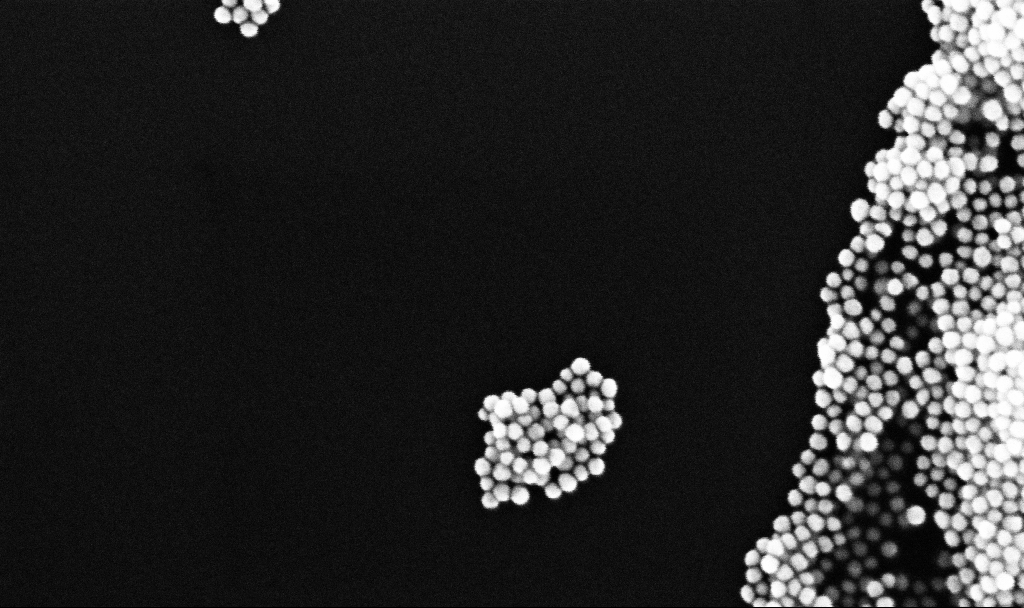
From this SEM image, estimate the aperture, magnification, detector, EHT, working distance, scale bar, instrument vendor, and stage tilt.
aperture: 30 µm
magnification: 298.11 K X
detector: InLens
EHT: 10 kV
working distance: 3.3 mm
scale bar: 200 nm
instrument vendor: Zeiss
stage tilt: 0°